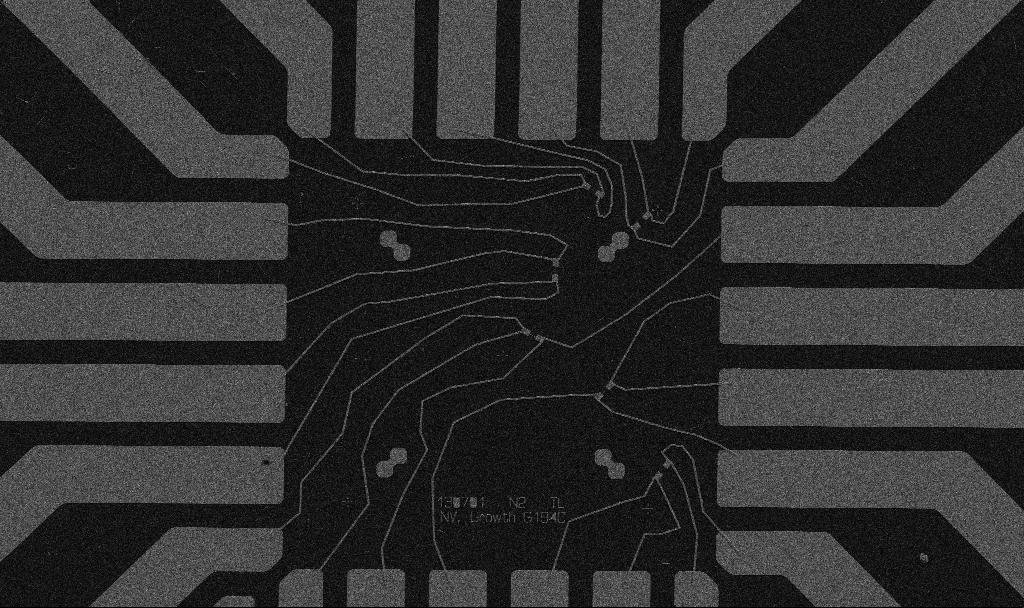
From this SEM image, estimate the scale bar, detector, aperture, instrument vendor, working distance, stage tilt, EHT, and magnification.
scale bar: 20000 nm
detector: SE2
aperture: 30 µm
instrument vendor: Zeiss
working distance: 10.7 mm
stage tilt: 0°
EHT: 5 kV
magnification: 1 K X